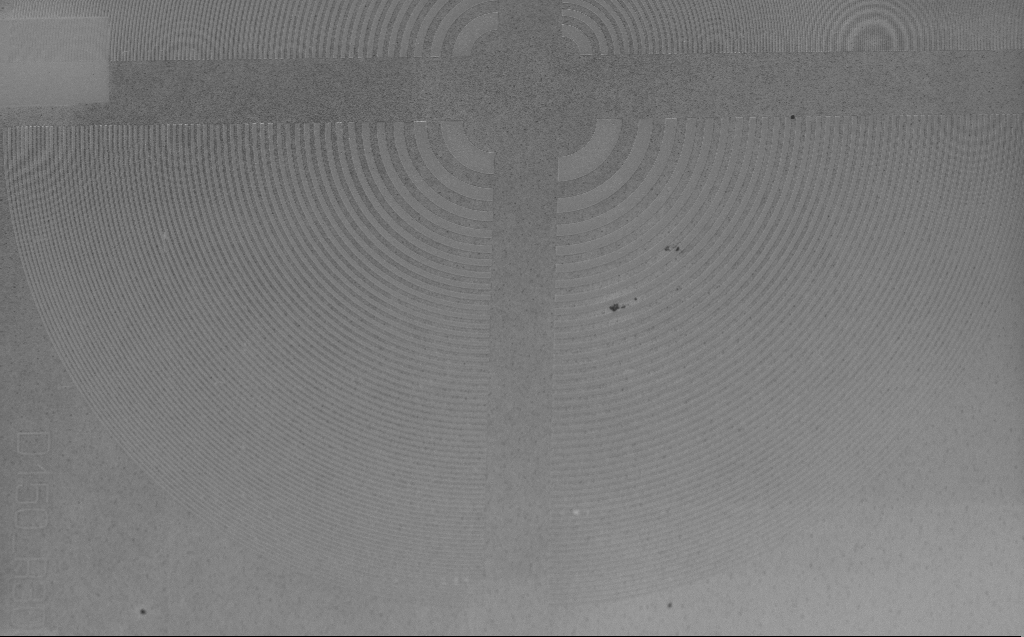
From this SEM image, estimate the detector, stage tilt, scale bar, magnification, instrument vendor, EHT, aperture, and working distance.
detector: InLens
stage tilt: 30°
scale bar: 10000 nm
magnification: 2.41 K X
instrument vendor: Zeiss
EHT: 5 kV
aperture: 30 µm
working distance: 4 mm